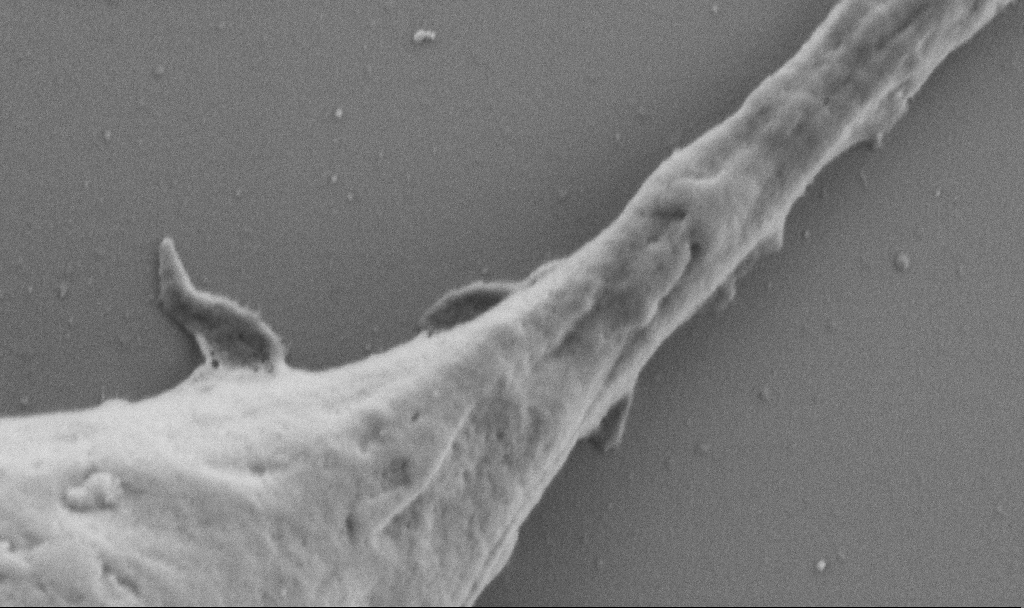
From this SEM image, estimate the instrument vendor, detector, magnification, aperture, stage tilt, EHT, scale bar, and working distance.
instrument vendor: Zeiss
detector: SE2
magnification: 50 K X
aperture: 30 µm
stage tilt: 0°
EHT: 1 kV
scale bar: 1000 nm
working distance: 6.9 mm